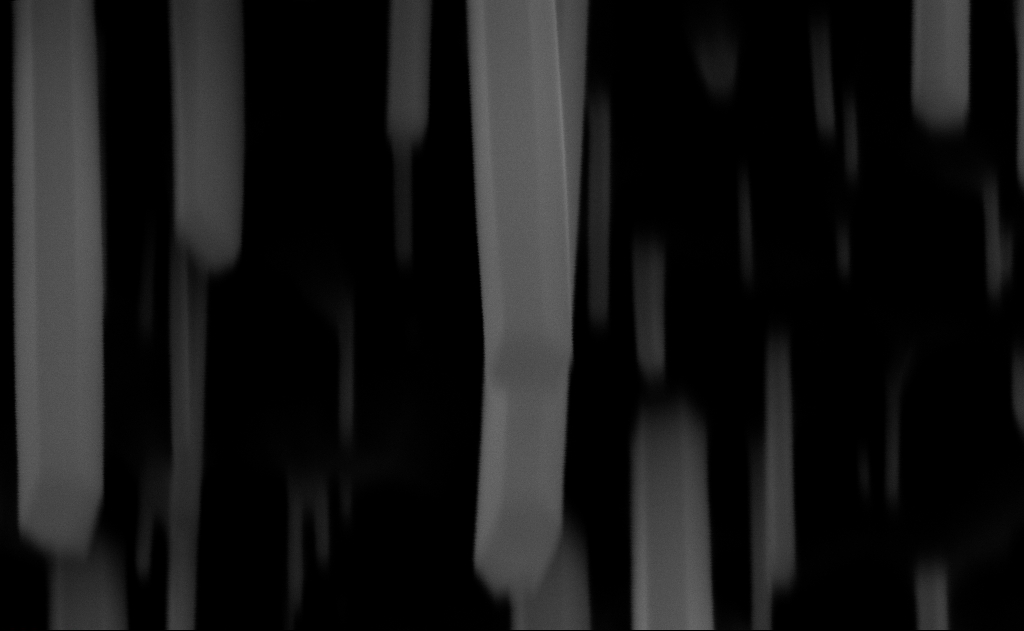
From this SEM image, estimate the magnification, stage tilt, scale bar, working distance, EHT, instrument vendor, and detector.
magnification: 200 K X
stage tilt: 0°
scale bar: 200 nm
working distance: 9 mm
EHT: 10 kV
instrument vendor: Zeiss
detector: InLens